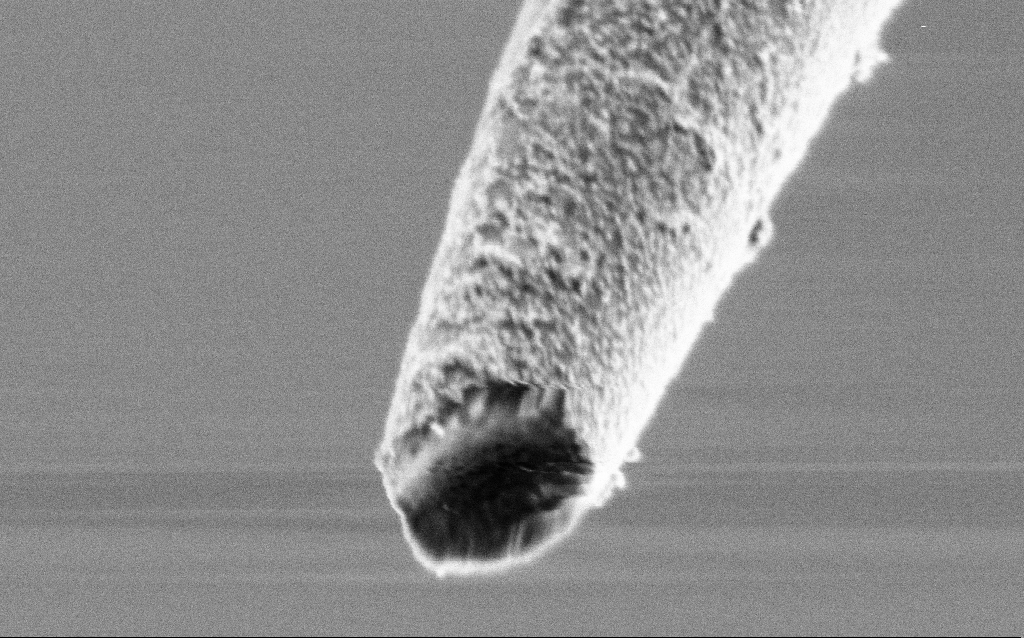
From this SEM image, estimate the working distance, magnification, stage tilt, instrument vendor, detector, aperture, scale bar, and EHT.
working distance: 6.7 mm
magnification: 100 K X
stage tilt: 45°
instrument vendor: Zeiss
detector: SE2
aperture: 30 µm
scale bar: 200 nm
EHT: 1 kV